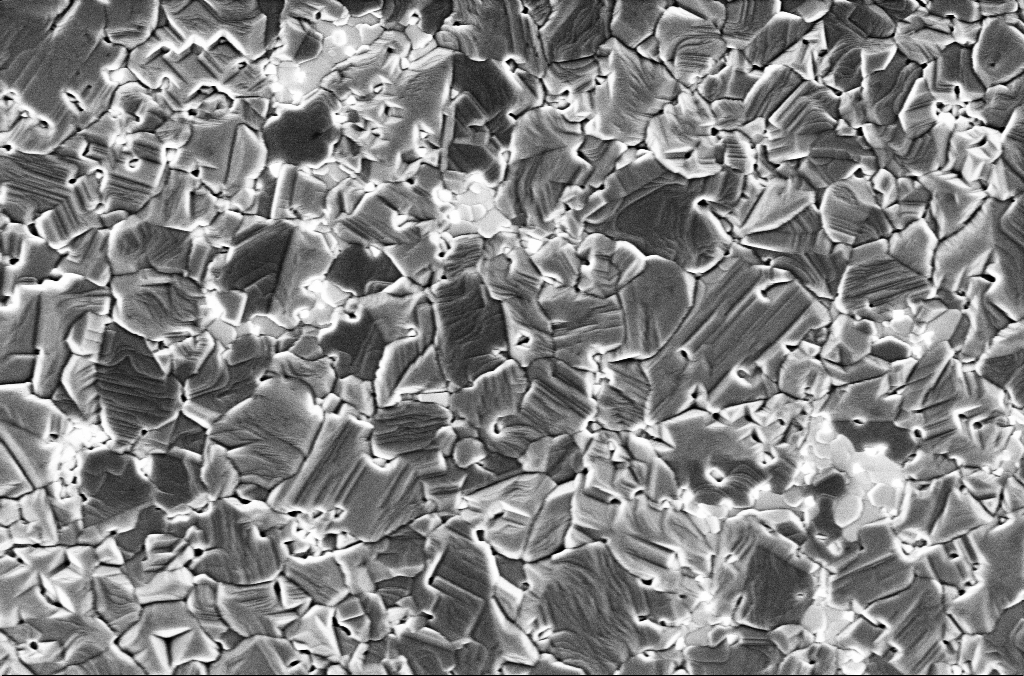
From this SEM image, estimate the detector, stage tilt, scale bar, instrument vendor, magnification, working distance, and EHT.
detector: InLens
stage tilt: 0°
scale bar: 1000 nm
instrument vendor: Zeiss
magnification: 42.28 K X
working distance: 3 mm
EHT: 2 kV